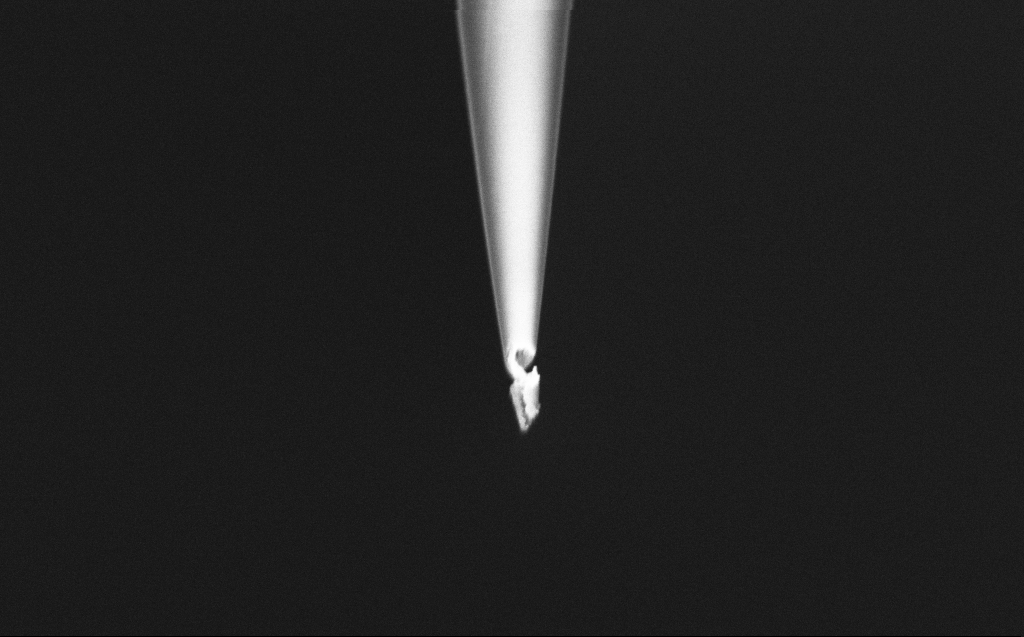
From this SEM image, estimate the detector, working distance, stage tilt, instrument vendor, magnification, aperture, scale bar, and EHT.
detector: InLens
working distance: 6 mm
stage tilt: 45°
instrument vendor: Zeiss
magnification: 50 K X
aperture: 30 µm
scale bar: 1000 nm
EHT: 2 kV